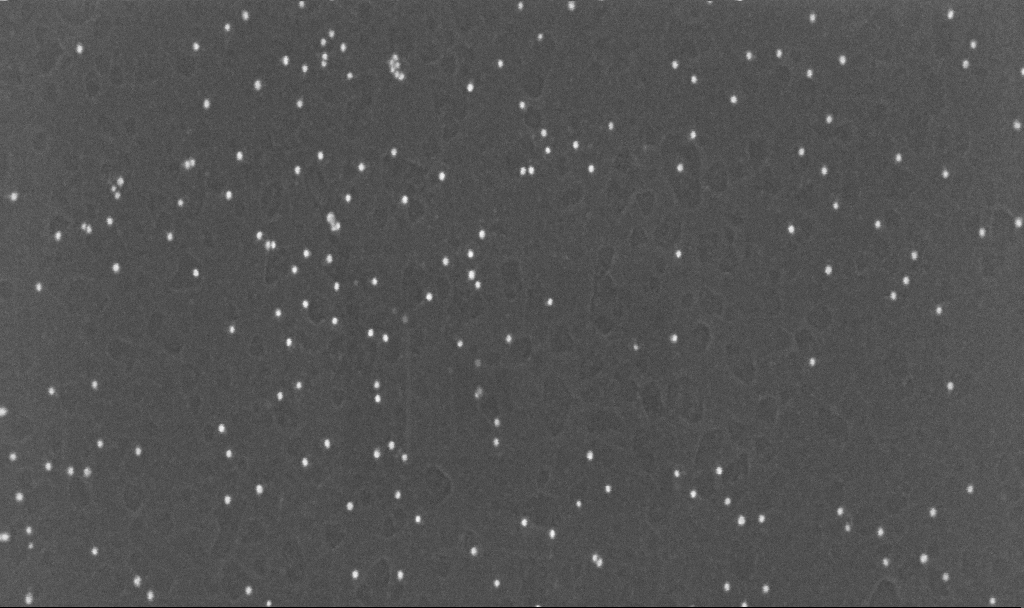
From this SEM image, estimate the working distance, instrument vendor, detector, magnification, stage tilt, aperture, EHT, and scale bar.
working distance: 3.1 mm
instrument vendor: Zeiss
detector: InLens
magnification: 200 K X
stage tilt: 0°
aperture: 30 µm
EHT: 10 kV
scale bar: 100 nm